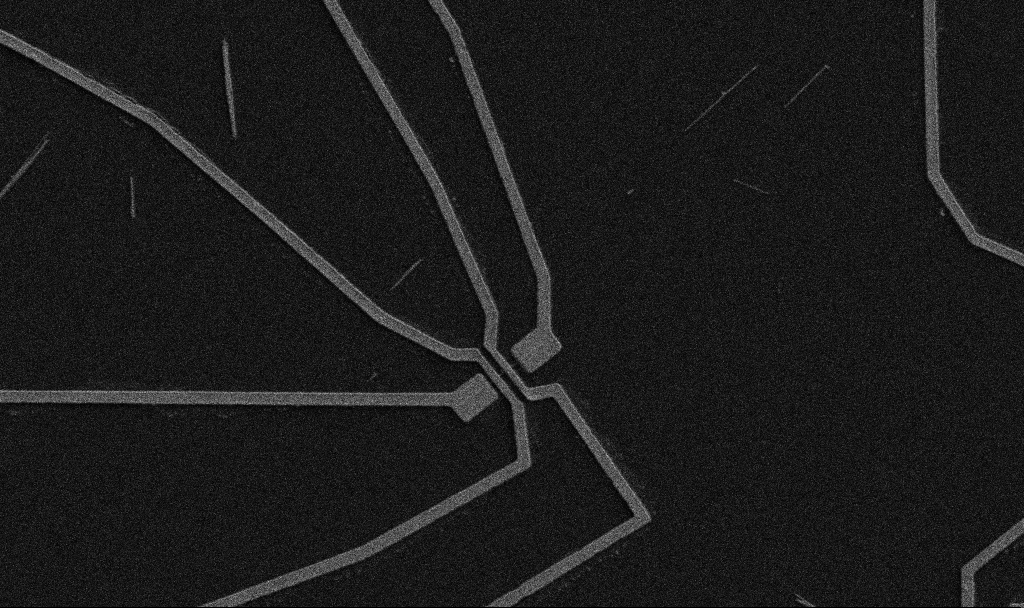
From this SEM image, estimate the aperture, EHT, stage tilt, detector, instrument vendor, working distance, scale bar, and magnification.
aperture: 30 µm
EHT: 5 kV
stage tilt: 0°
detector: SE2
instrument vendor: Zeiss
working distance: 10.7 mm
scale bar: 10000 nm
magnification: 5 K X